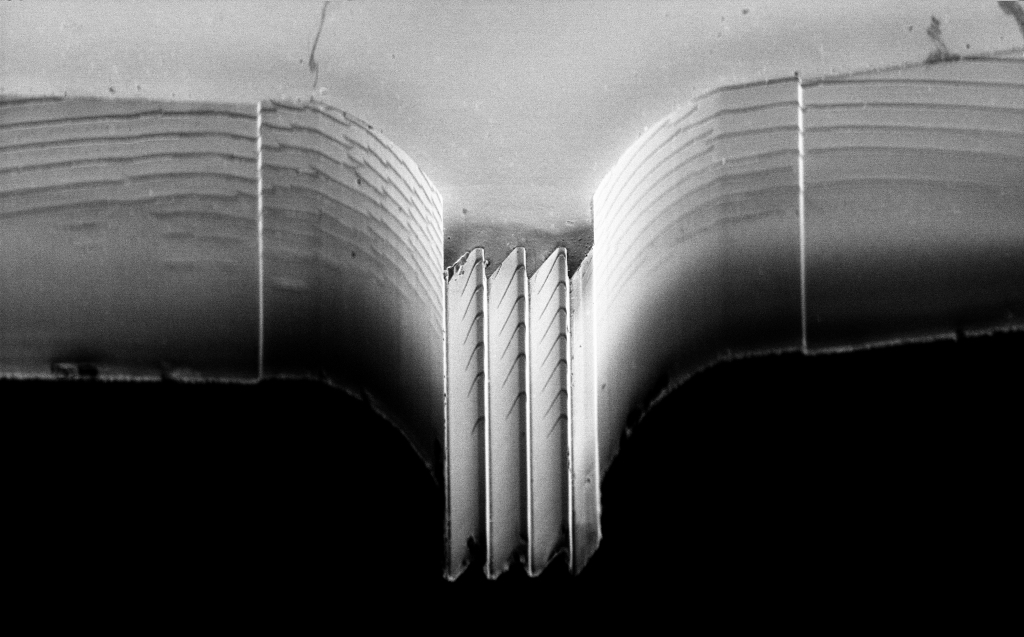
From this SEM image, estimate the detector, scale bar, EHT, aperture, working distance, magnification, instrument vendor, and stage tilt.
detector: InLens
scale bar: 10000 nm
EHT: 3 kV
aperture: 30 µm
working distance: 4 mm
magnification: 1.35 K X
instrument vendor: Zeiss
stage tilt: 45°